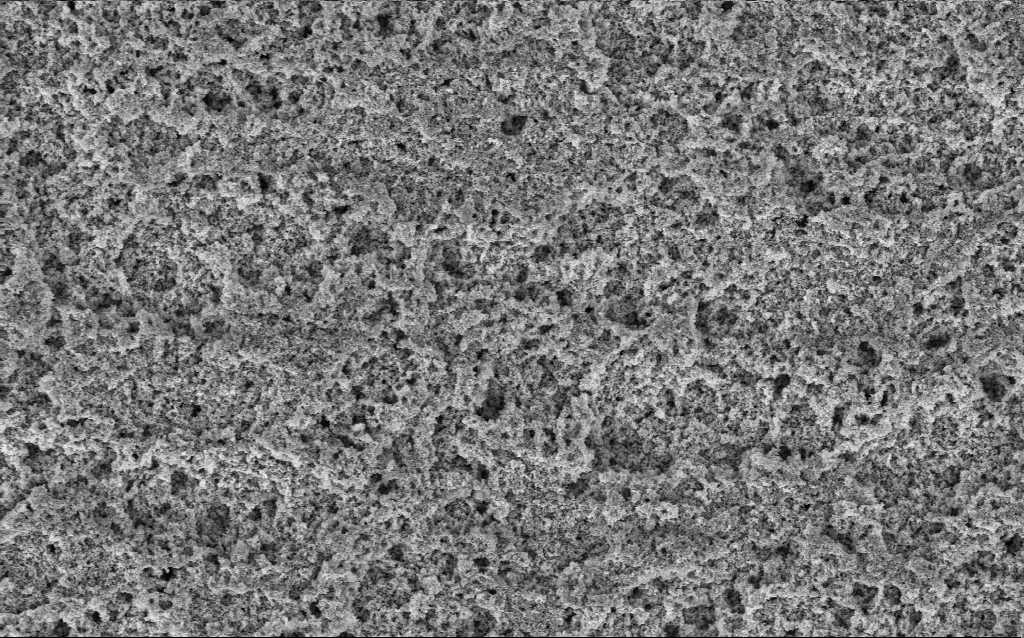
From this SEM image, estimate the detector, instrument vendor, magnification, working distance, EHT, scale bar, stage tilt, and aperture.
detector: InLens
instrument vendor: Zeiss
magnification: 20.87 K X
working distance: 10 mm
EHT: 3 kV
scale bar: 1000 nm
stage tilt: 0°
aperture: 30 µm